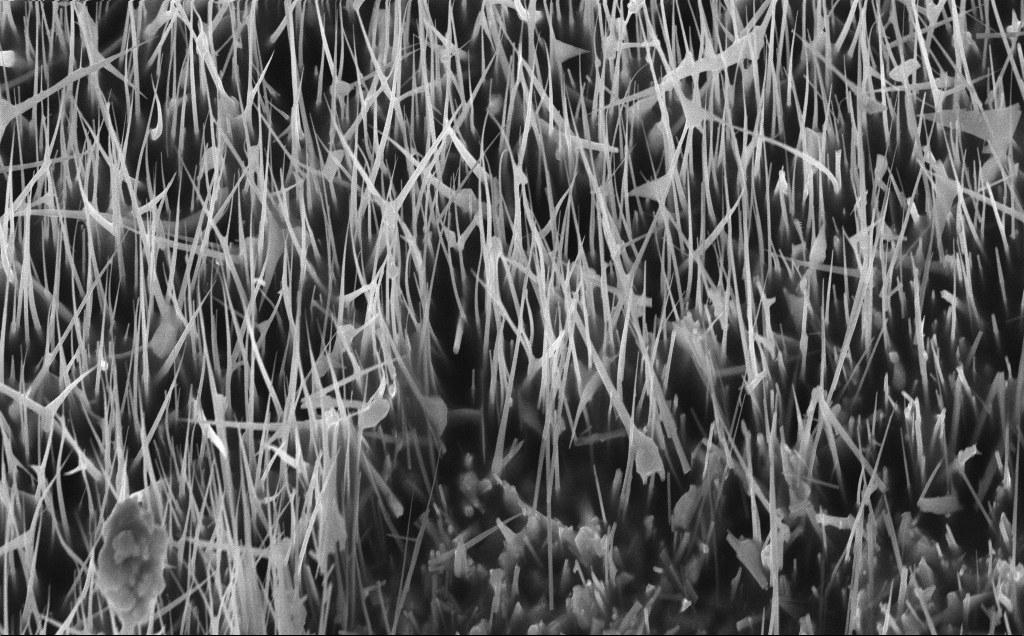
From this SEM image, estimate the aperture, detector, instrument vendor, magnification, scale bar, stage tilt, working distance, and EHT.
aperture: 30 µm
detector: InLens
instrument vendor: Zeiss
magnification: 17 K X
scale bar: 2000 nm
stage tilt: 30°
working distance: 7 mm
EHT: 10 kV